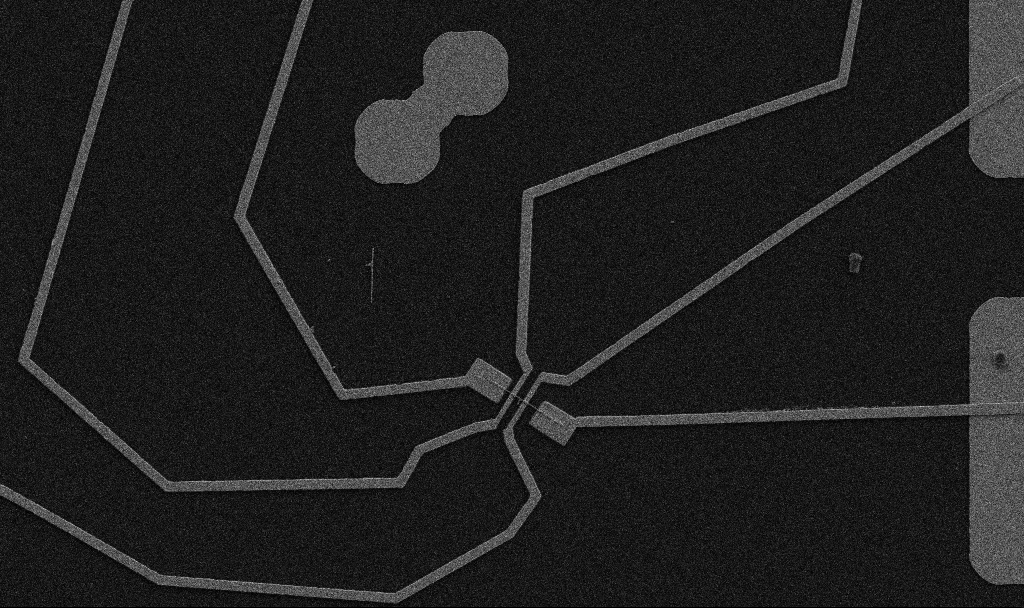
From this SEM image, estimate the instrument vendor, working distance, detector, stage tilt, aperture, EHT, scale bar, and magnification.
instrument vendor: Zeiss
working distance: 10.7 mm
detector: SE2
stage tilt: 0°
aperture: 30 µm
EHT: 5 kV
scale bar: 10000 nm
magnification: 5 K X